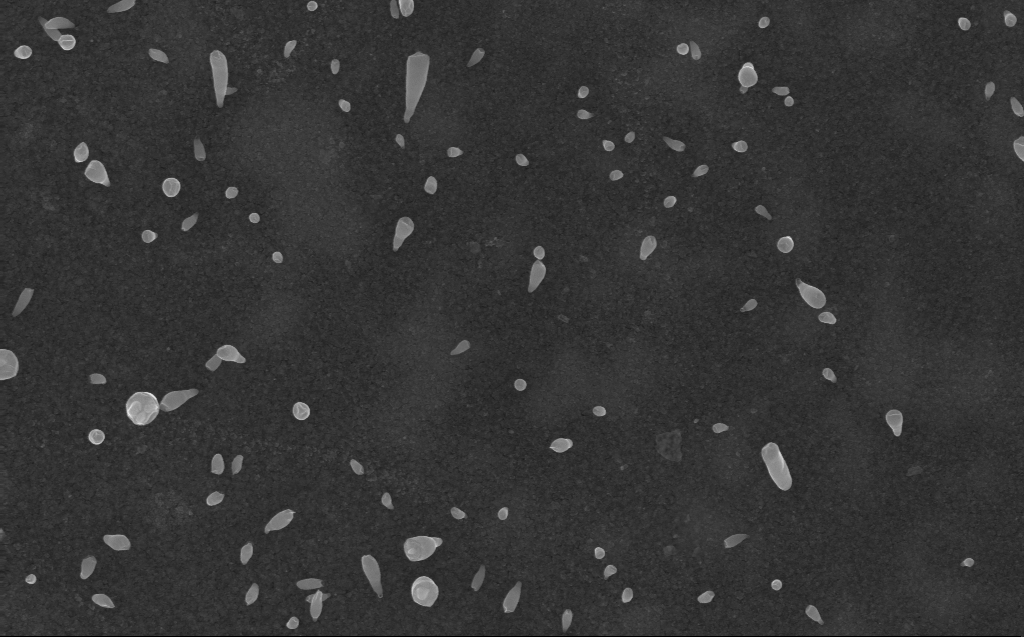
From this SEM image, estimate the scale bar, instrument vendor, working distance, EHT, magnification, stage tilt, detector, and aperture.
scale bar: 1000 nm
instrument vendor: Zeiss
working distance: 3 mm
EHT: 10 kV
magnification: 50 K X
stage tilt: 0°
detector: InLens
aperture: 30 µm